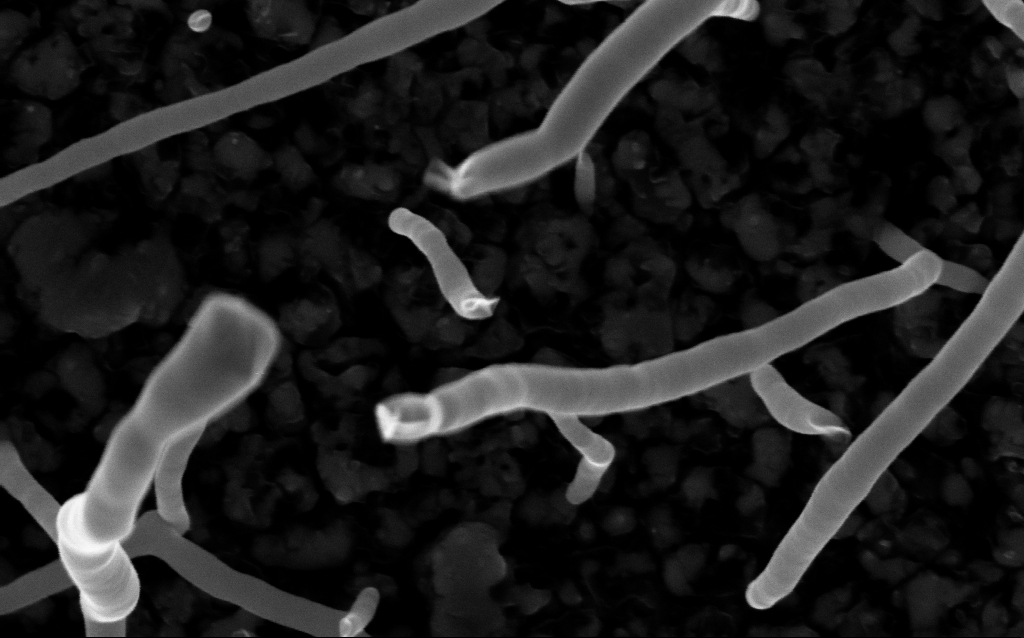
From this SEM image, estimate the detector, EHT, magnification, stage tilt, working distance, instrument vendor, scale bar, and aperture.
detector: InLens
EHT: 5 kV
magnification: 200 K X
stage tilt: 0°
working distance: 2.2 mm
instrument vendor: Zeiss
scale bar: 100 nm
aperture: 30 µm